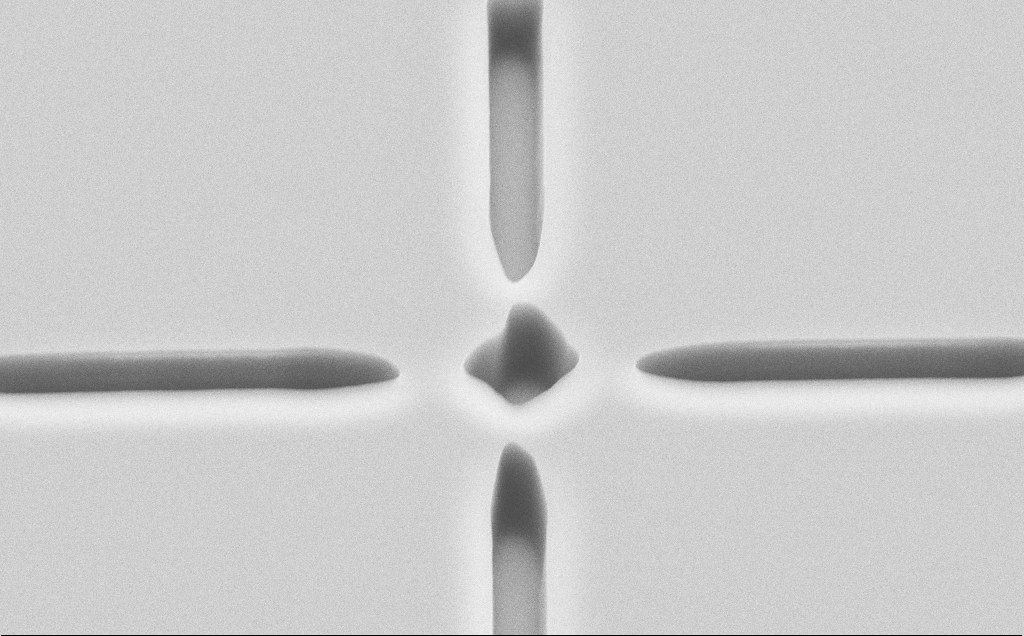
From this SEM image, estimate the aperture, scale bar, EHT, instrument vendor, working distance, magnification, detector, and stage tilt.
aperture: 30 µm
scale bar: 2000 nm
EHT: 10 kV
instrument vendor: Zeiss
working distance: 6 mm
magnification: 11.26 K X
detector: SE2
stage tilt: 45°